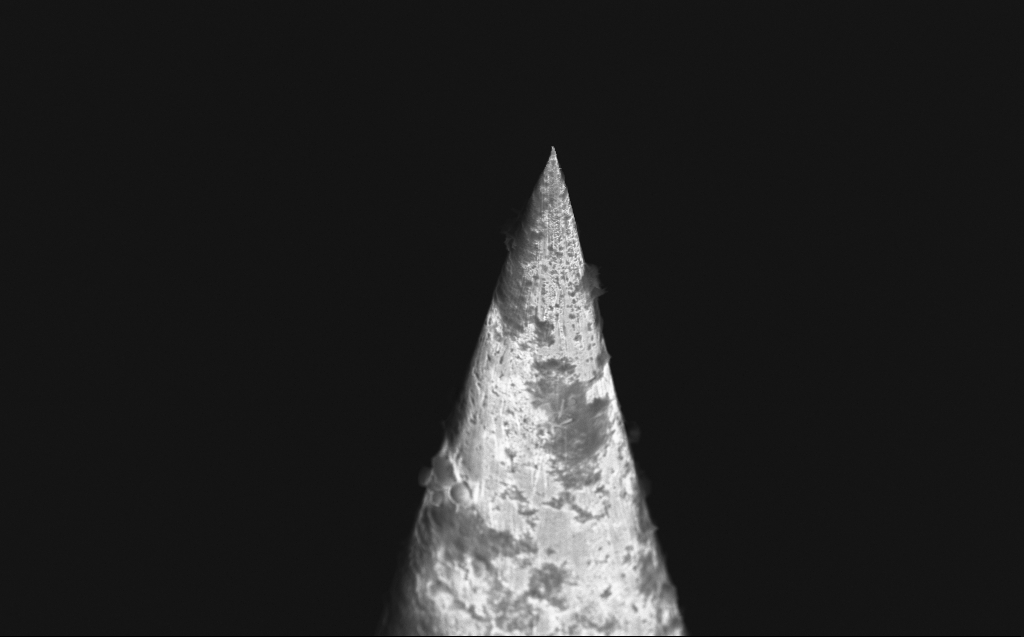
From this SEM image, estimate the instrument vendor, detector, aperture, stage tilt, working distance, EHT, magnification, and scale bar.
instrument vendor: Zeiss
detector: InLens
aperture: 30 µm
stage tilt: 40°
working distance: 4 mm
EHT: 10 kV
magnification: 5.54 K X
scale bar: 10000 nm